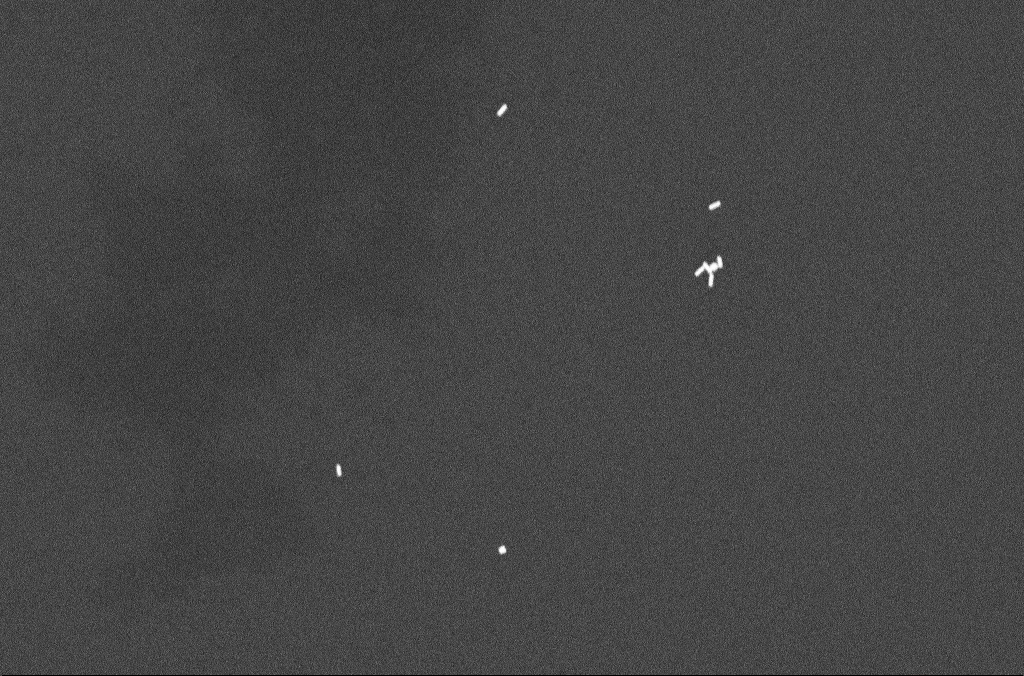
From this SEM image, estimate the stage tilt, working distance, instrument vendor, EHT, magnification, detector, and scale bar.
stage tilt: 0°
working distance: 4.8 mm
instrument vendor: Zeiss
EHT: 10 kV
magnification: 67.76 K X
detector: HDAsB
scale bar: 200 nm